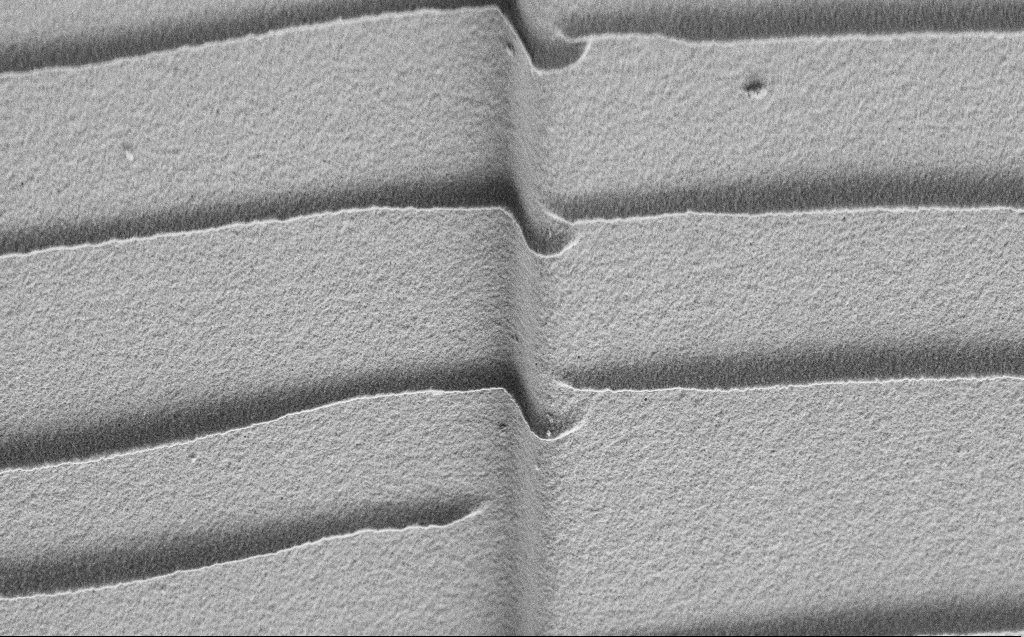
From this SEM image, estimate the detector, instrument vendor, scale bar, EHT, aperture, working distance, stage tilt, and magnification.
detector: SE2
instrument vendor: Zeiss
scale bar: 2000 nm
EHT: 3 kV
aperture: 30 µm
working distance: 4 mm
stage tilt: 45°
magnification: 10.64 K X